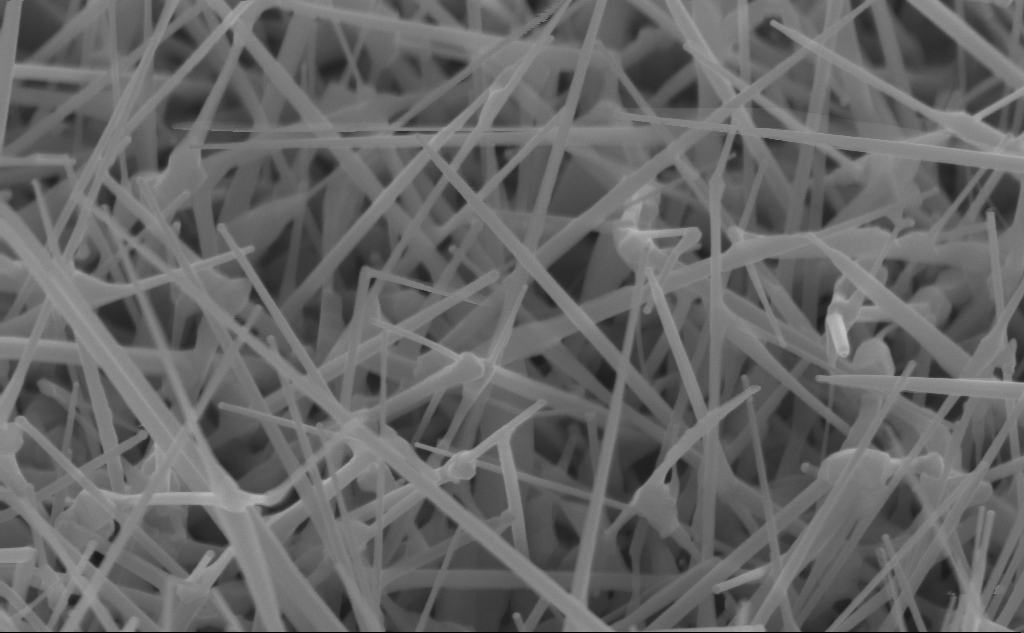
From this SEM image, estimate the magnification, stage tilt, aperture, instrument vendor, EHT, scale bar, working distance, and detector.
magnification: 81.68 K X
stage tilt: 45°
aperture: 30 µm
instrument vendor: Zeiss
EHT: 10 kV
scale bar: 200 nm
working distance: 4 mm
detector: InLens